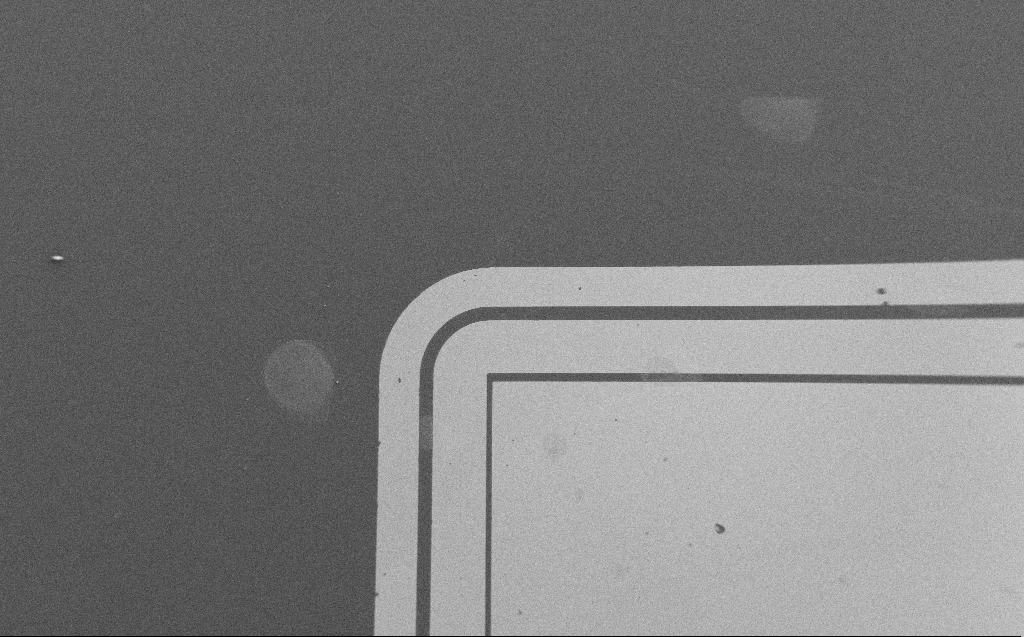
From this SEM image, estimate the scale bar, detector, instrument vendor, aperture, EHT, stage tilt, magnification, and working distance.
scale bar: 100000 nm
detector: SE2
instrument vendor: Zeiss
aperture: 30 µm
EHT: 1.2 kV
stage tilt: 0°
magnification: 0.196 K X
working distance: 6 mm